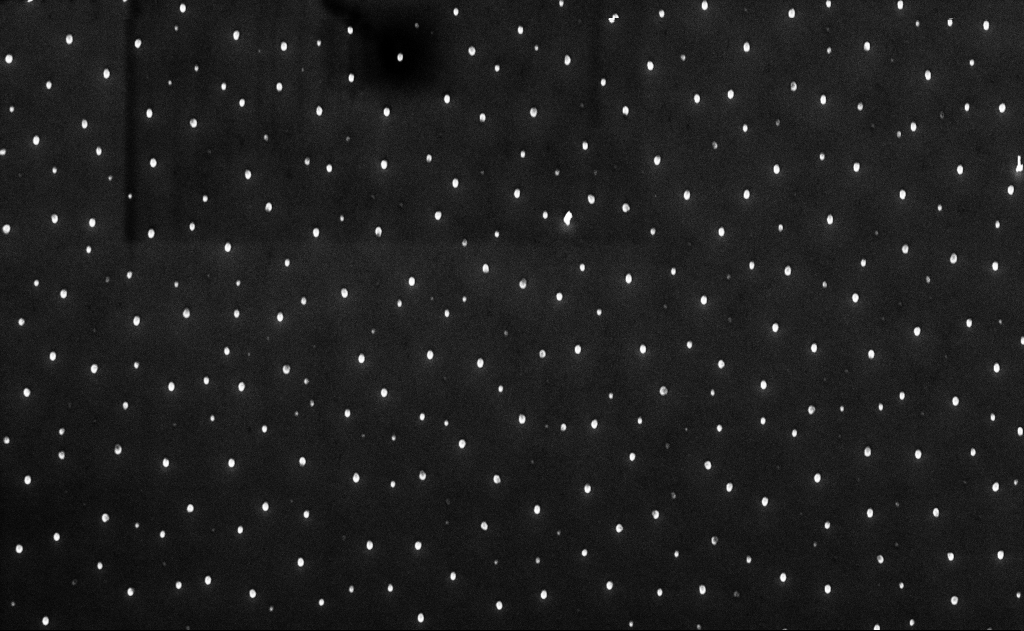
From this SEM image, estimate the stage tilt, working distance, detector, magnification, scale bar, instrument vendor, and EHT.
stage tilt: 0°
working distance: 10 mm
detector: InLens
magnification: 5 K X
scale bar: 10000 nm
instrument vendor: Zeiss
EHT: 10 kV